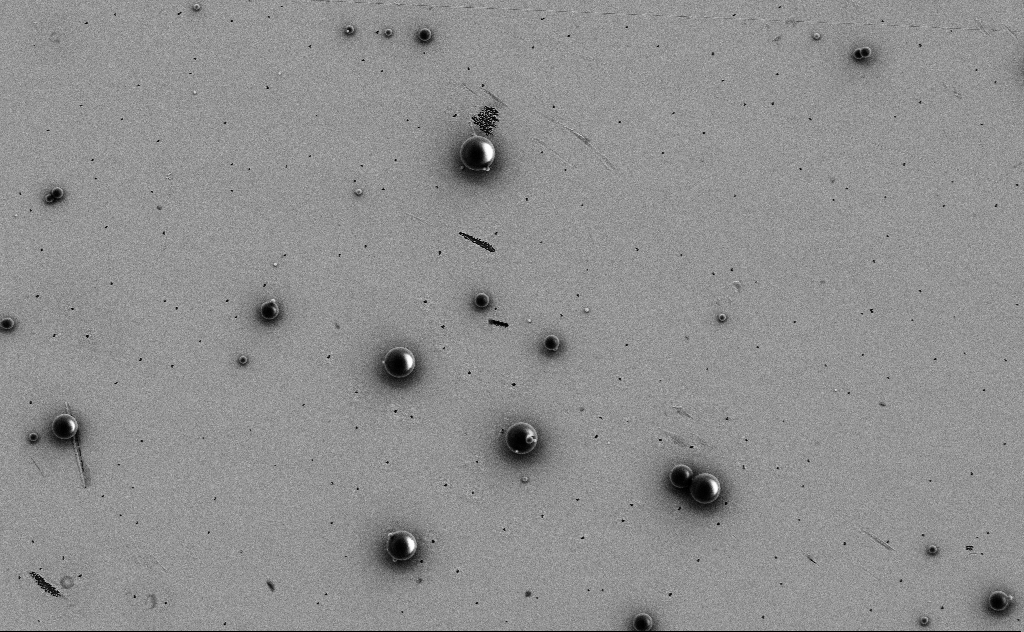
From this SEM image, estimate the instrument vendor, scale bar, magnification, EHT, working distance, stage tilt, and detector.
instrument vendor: Zeiss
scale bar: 10000 nm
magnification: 6.76 K X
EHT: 3 kV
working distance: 10 mm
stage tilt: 0°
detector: SE2